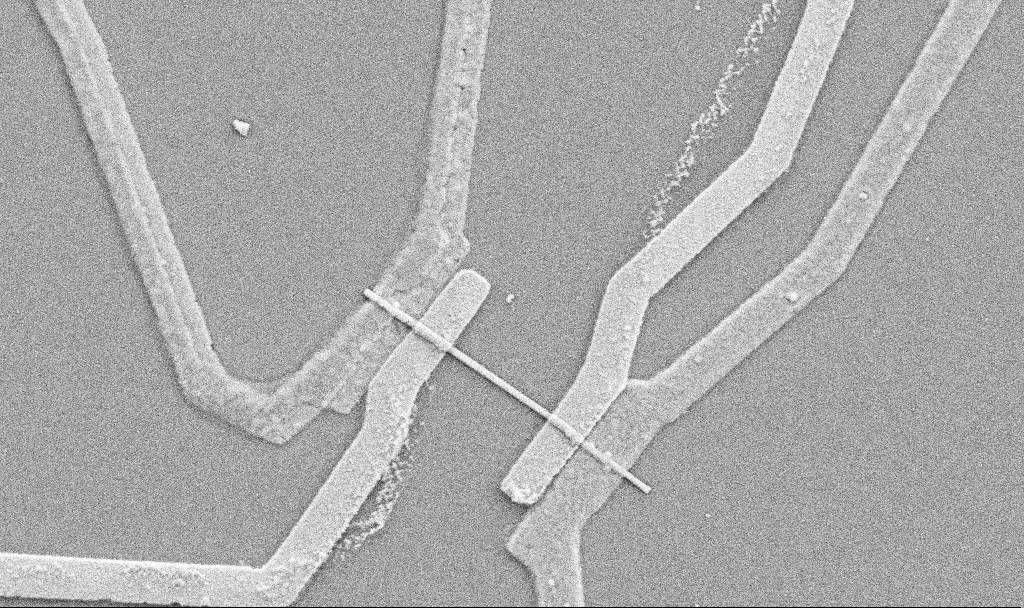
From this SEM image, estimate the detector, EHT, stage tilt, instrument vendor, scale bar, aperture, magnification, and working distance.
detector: SE2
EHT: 5 kV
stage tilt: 0°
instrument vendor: Zeiss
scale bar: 1000 nm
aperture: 30 µm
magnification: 20 K X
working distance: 10.7 mm